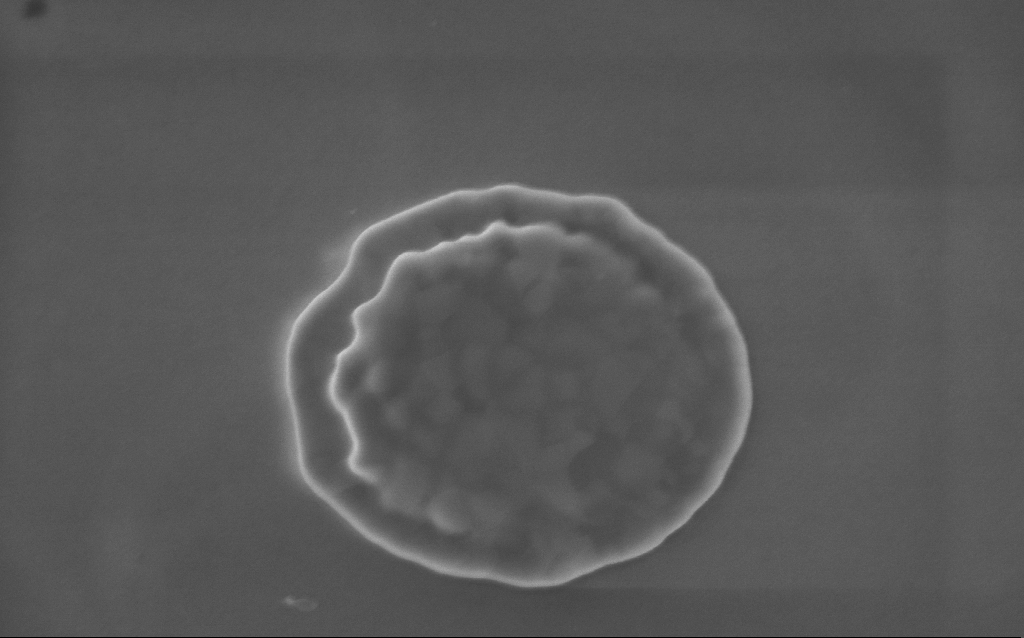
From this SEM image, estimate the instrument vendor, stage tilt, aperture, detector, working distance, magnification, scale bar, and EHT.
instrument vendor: Zeiss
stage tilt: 0°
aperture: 30 µm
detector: InLens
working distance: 3 mm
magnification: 88.38 K X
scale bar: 200 nm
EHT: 5 kV